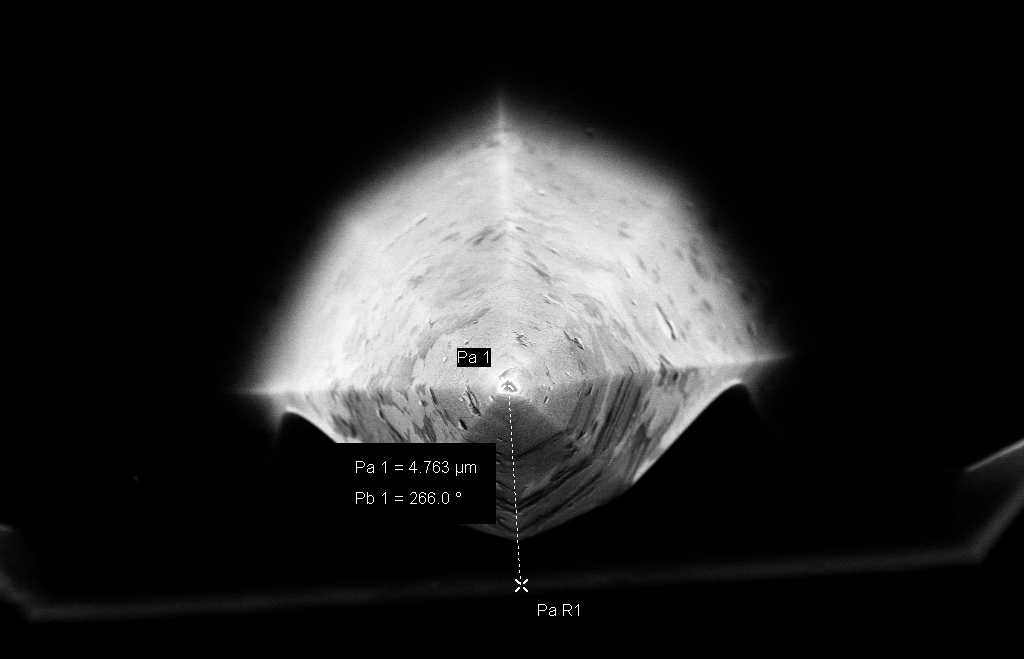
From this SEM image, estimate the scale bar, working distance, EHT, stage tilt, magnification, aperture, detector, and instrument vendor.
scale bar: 1000 nm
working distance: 8 mm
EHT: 10 kV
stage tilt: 0°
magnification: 15.61 K X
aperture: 30 µm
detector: InLens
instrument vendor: Zeiss